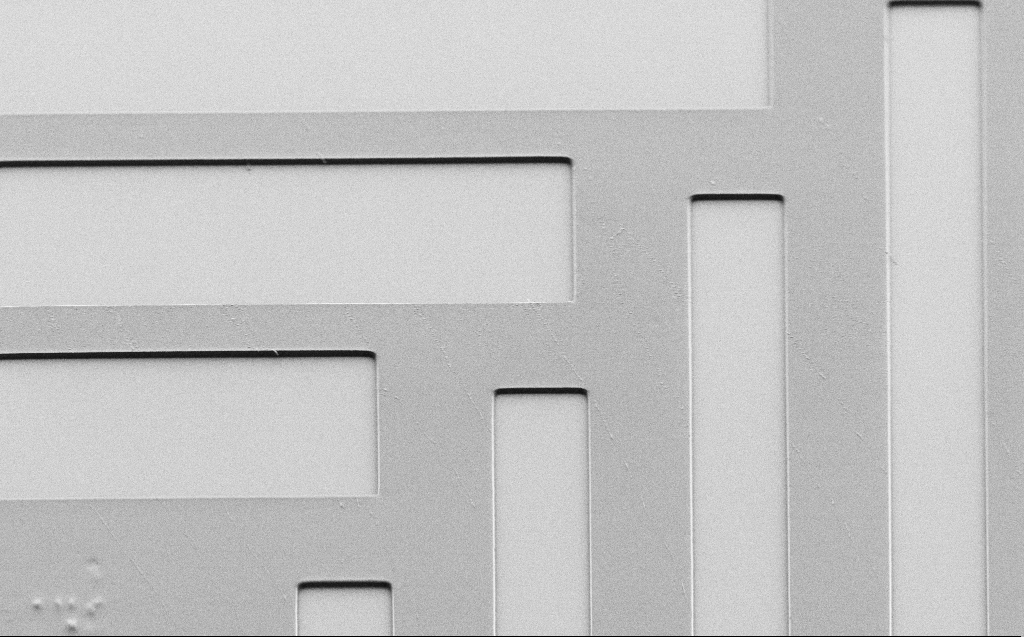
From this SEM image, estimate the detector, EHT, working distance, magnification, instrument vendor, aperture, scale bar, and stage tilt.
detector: SE2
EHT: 1.7 kV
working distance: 6 mm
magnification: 0.969 K X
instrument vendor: Zeiss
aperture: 30 µm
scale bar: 20000 nm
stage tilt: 45°